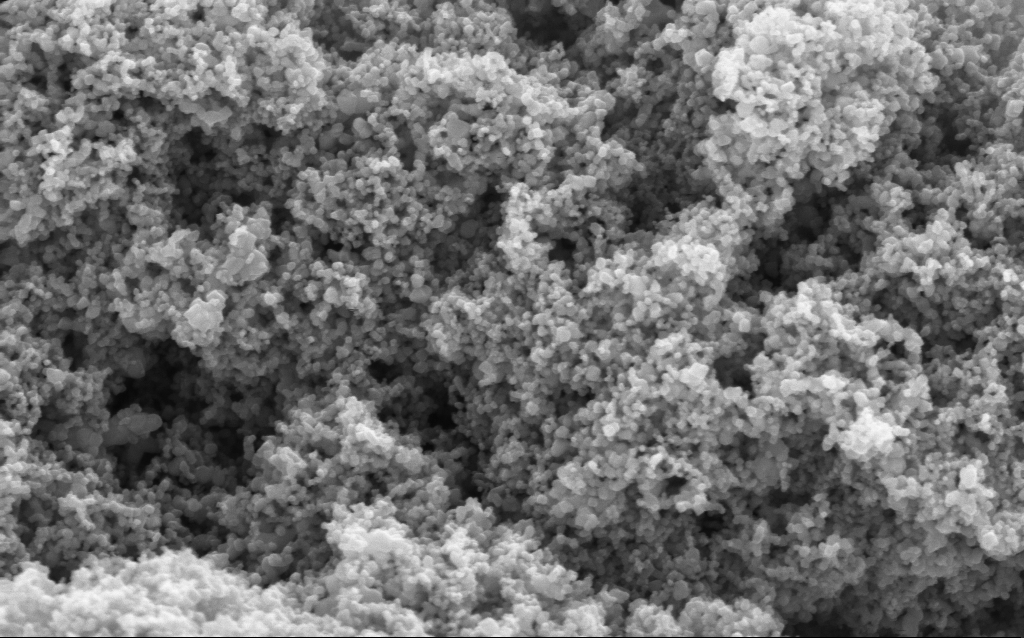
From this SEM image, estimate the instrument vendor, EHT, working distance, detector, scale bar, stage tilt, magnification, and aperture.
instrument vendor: Zeiss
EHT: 5 kV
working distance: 4.5 mm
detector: InLens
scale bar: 200 nm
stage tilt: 0°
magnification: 114.65 K X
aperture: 30 µm